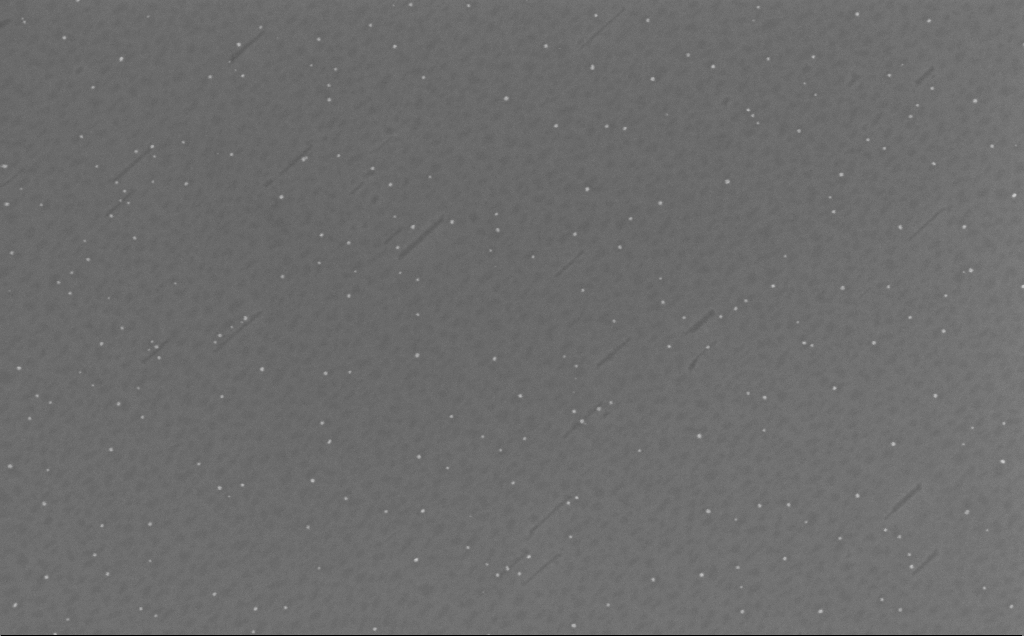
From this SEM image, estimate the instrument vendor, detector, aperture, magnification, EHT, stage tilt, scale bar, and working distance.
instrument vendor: Zeiss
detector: InLens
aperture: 30 µm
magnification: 173.55 K X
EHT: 10 kV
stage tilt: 0°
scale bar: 100 nm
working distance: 6 mm